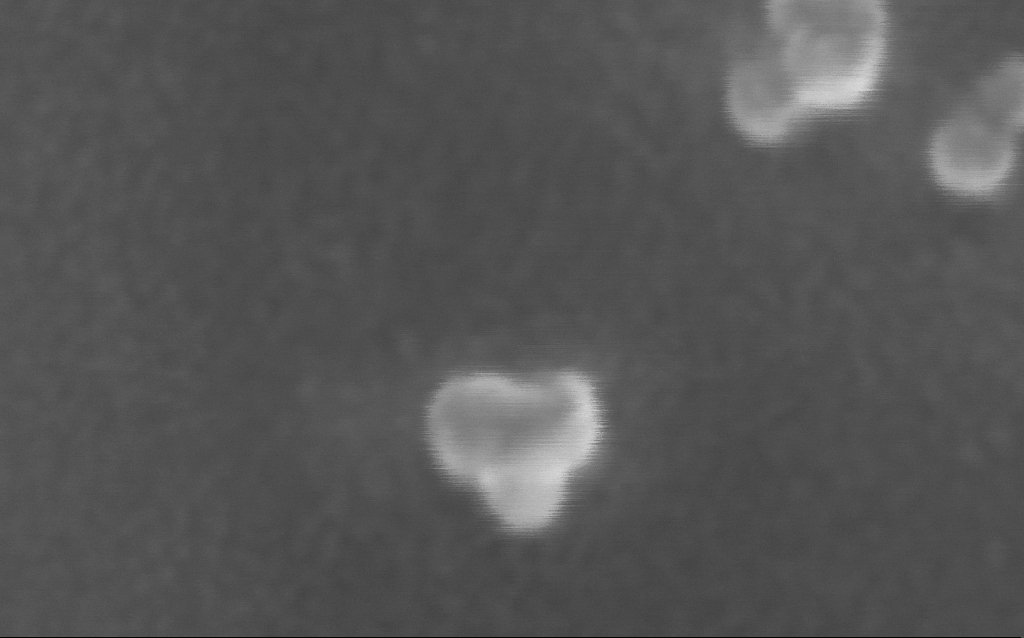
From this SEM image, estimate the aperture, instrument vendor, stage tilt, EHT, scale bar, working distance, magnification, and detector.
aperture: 30 µm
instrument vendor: Zeiss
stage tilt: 0°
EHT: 10 kV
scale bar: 20 nm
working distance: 5 mm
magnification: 1905.41 K X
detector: InLens